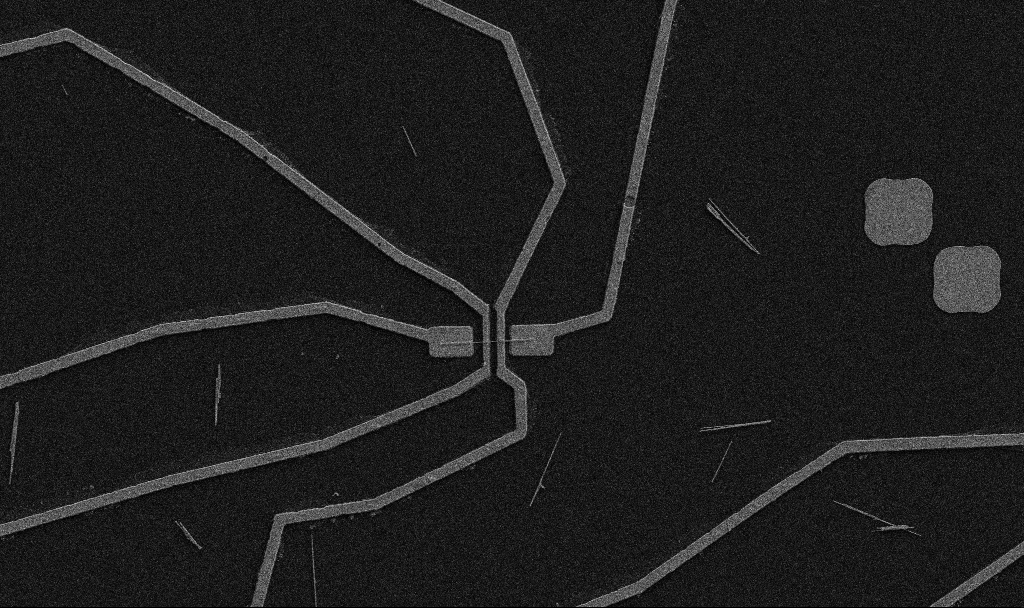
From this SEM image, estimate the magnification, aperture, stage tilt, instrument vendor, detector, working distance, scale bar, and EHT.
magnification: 5 K X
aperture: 30 µm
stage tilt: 0°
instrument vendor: Zeiss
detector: SE2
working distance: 10.7 mm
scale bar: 10000 nm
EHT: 5 kV